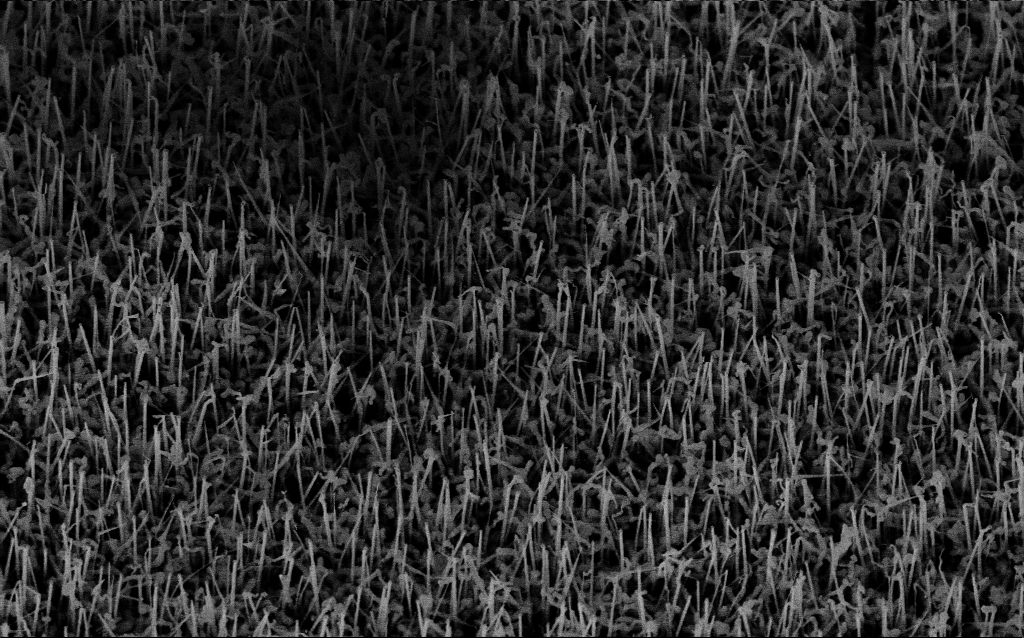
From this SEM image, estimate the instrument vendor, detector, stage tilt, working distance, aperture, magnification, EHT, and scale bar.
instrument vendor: Zeiss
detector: InLens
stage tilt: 35°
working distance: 7.3 mm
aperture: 30 µm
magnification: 36.43 K X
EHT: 10 kV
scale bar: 1000 nm